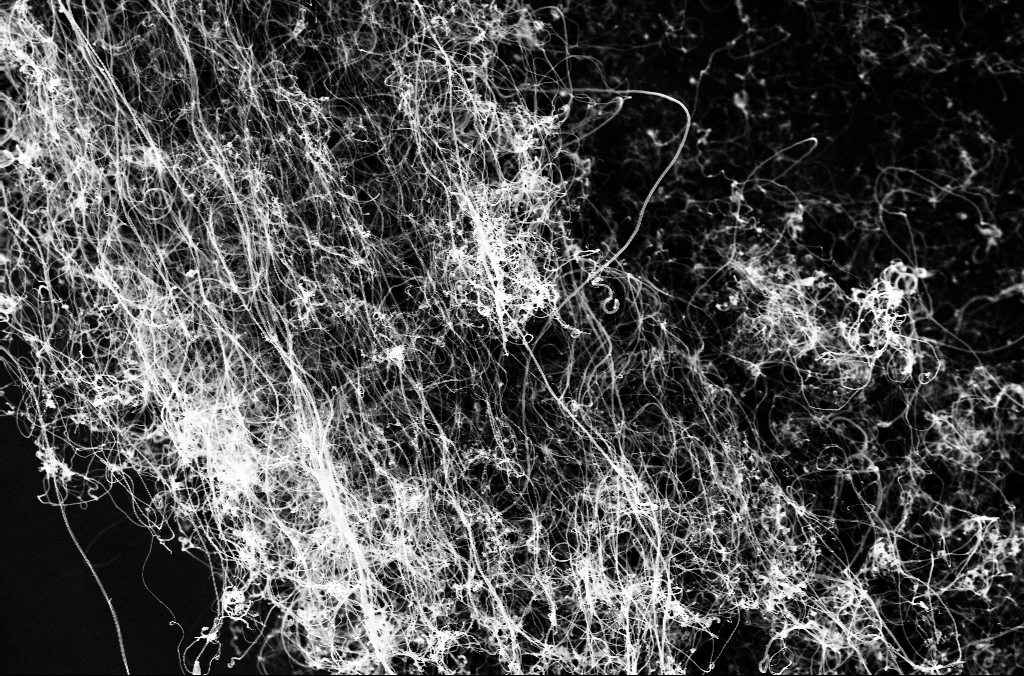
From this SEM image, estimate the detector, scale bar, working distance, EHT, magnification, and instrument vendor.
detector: InLens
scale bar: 2000 nm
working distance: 3.3 mm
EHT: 10 kV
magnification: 10 K X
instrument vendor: Zeiss